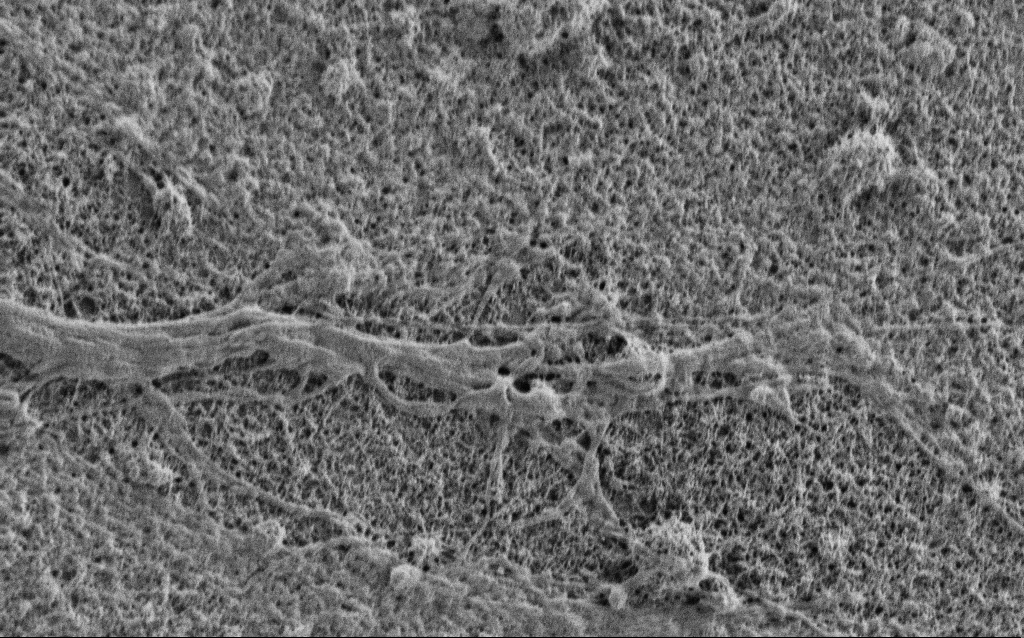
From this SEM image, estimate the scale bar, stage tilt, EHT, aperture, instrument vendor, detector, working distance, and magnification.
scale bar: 1000 nm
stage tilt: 0°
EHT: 0.9 kV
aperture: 30 µm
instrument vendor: Zeiss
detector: SE2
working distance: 5.3 mm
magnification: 15 K X